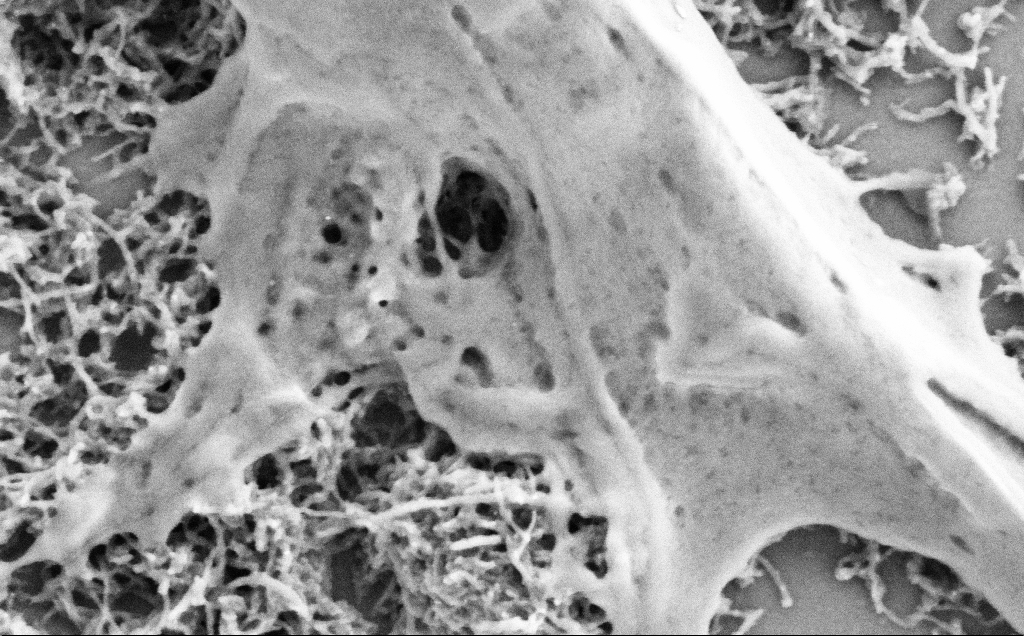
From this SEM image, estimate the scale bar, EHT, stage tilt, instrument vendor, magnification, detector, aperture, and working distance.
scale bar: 200 nm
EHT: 2 kV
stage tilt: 0°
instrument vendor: Zeiss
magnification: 75 K X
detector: SE2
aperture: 30 µm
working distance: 7.1 mm